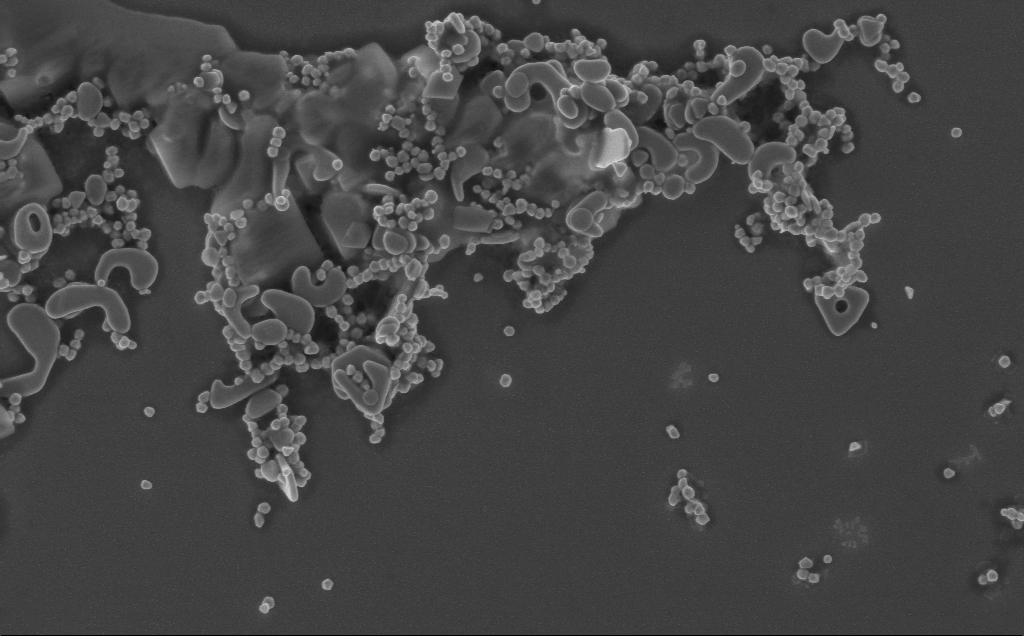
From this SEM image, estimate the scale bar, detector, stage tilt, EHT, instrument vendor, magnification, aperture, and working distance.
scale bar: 2000 nm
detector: InLens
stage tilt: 0°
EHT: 5 kV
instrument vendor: Zeiss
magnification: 28.84 K X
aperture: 30 µm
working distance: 3 mm